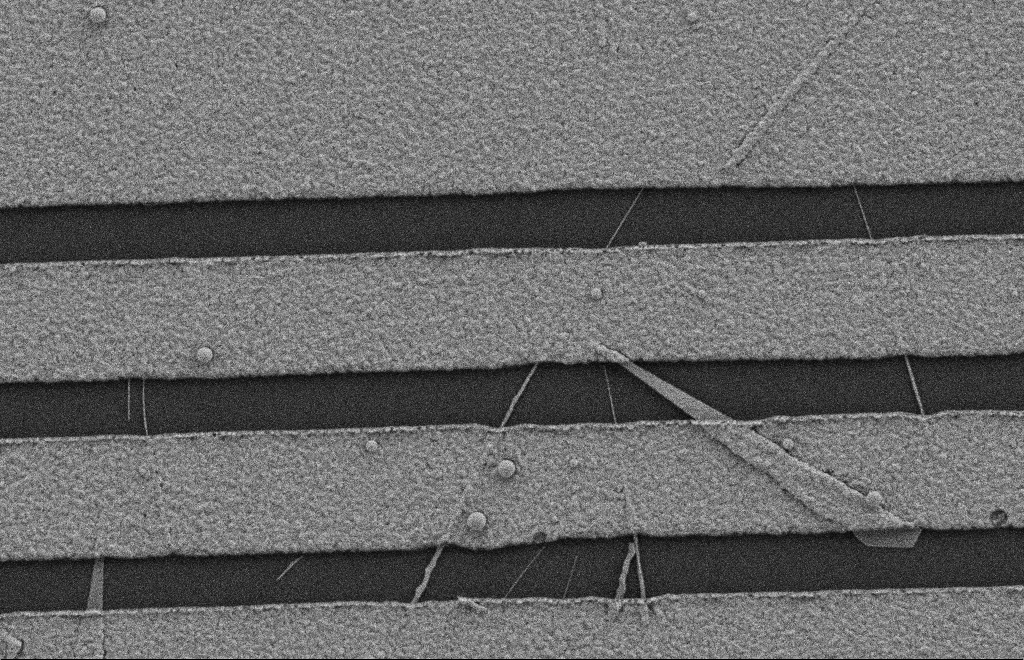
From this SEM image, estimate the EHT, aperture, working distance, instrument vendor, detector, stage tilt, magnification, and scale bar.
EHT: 2 kV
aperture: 20 µm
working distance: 9 mm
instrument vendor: Zeiss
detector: SE2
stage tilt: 0°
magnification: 16.05 K X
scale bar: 2000 nm